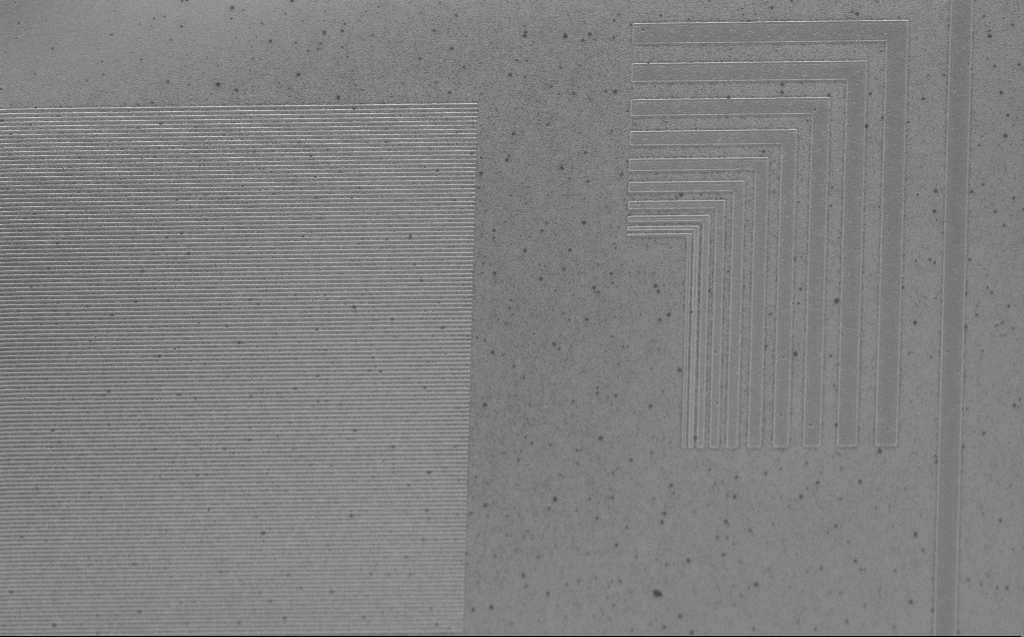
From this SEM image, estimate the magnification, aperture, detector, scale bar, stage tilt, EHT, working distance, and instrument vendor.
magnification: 7.96 K X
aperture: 30 µm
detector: InLens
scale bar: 2000 nm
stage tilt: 30°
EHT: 5 kV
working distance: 4 mm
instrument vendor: Zeiss